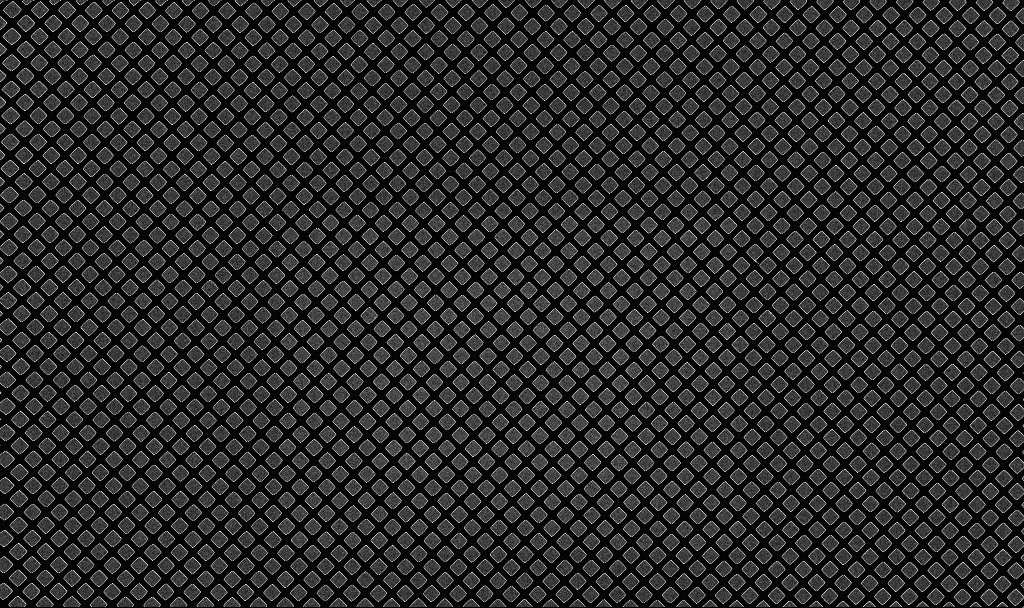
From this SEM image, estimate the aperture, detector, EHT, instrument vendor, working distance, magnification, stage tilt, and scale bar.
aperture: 30 µm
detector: InLens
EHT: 3 kV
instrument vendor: Zeiss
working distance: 7.7 mm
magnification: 1.15 K X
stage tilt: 0°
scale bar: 20000 nm